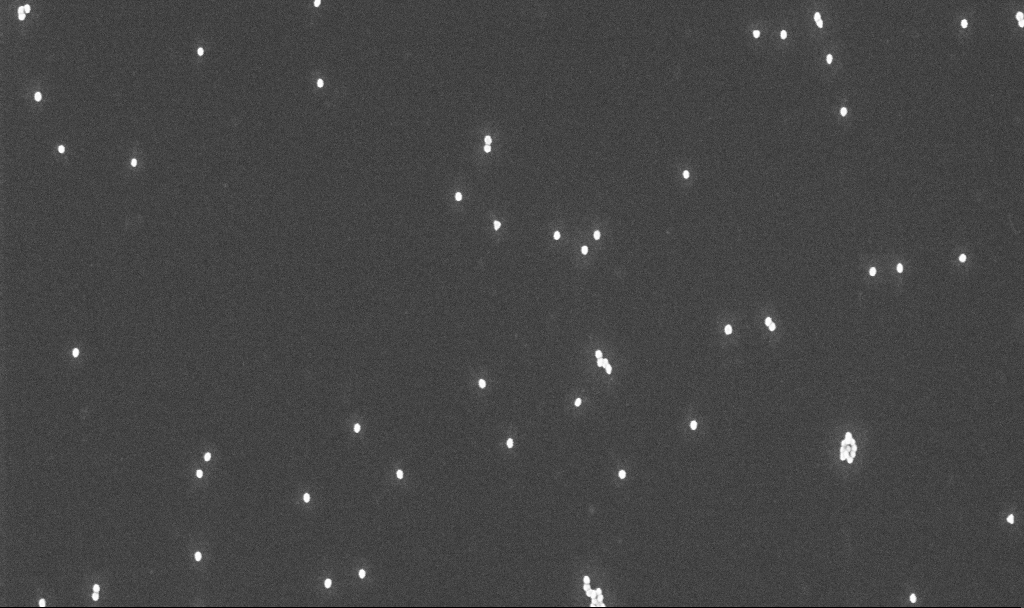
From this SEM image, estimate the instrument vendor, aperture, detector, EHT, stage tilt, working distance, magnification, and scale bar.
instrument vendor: Zeiss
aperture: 30 µm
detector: InLens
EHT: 10 kV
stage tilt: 0°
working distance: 4.8 mm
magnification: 100 K X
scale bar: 200 nm